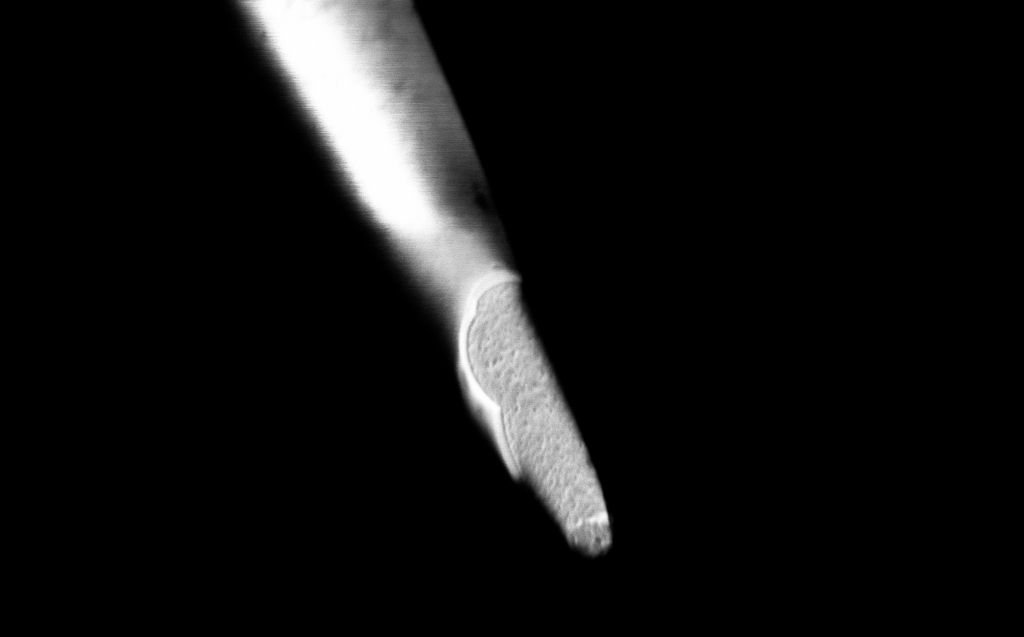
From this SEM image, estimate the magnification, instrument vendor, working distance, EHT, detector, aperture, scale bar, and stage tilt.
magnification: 50 K X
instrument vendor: Zeiss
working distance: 4 mm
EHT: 0.8 kV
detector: InLens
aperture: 30 µm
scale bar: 1000 nm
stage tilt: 45°